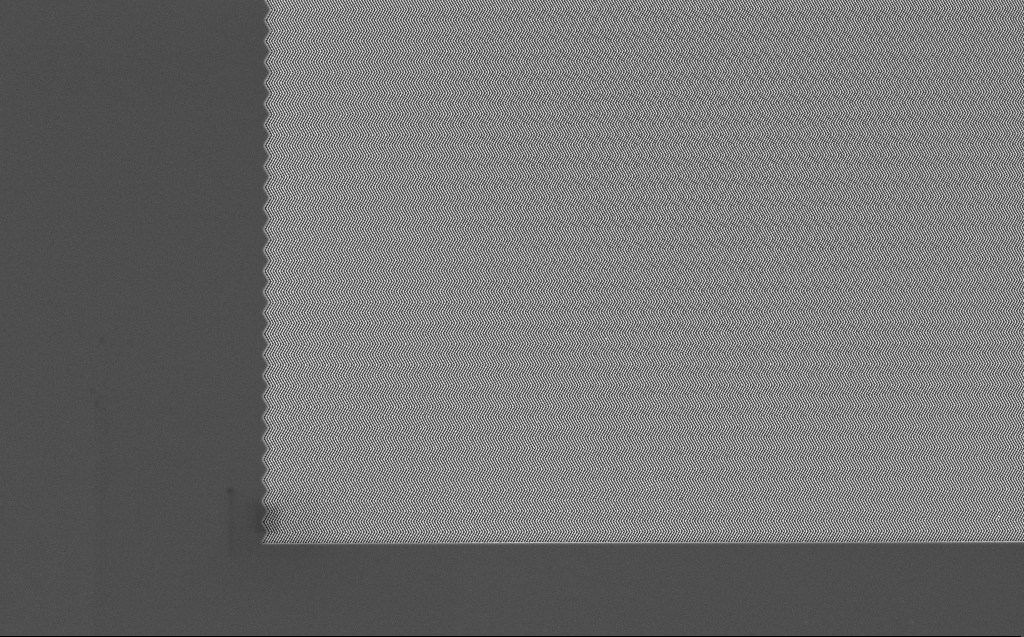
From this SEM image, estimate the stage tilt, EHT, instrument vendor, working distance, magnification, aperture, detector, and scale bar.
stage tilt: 0°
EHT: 5 kV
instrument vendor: Zeiss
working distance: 7 mm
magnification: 3.87 K X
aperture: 30 µm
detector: InLens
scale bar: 10000 nm